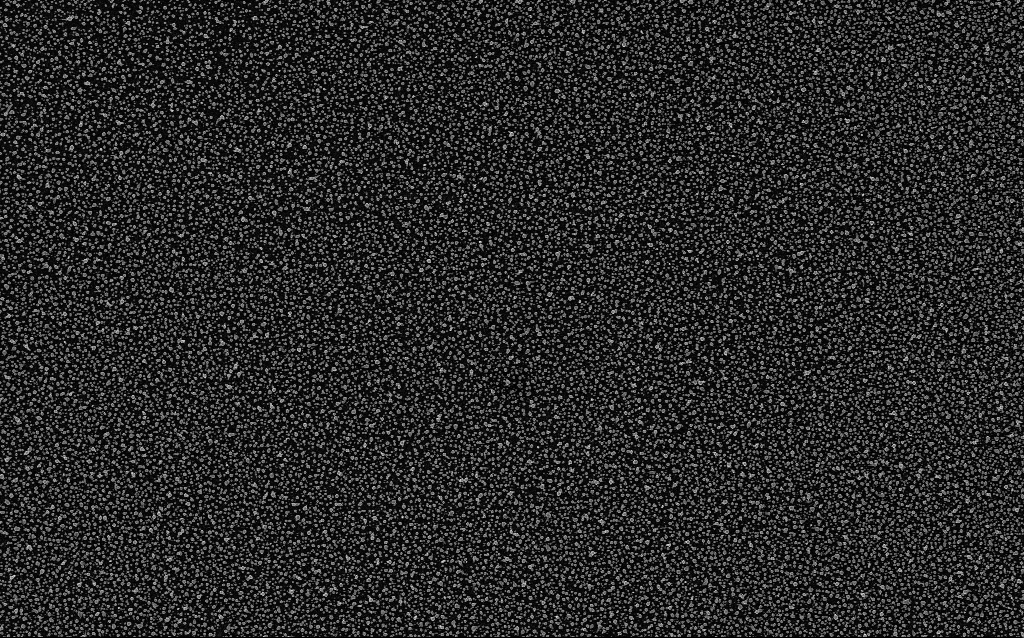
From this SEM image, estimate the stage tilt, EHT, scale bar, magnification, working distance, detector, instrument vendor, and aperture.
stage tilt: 0°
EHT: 5 kV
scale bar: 2000 nm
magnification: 10 K X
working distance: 2.1 mm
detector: InLens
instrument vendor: Zeiss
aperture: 30 µm